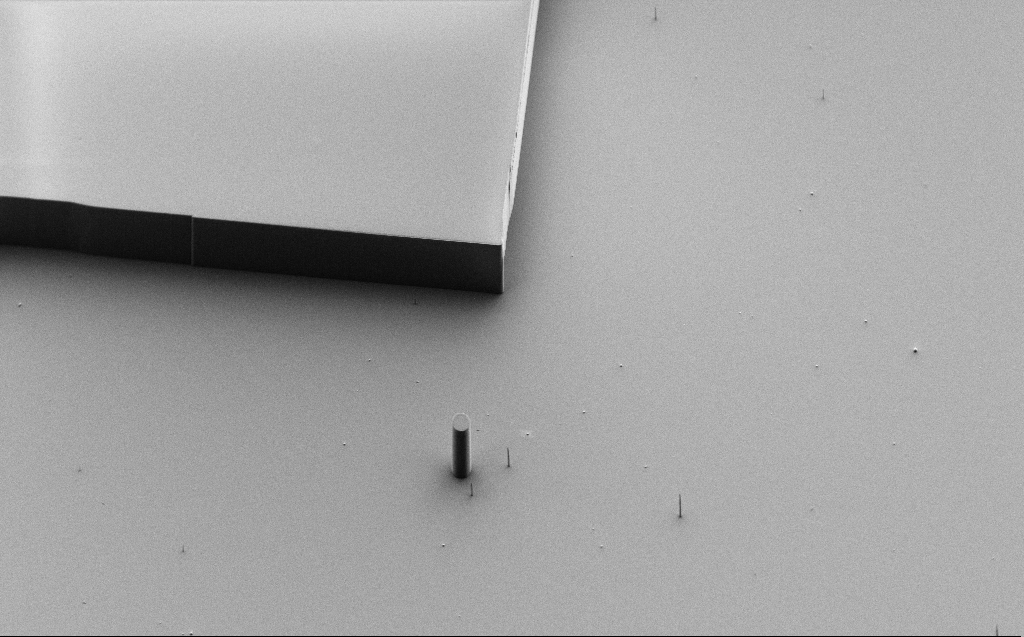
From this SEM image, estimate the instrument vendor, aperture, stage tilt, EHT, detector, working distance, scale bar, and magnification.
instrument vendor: Zeiss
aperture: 30 µm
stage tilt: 45°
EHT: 1.1 kV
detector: SE2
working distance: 7 mm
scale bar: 10000 nm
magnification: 1.34 K X